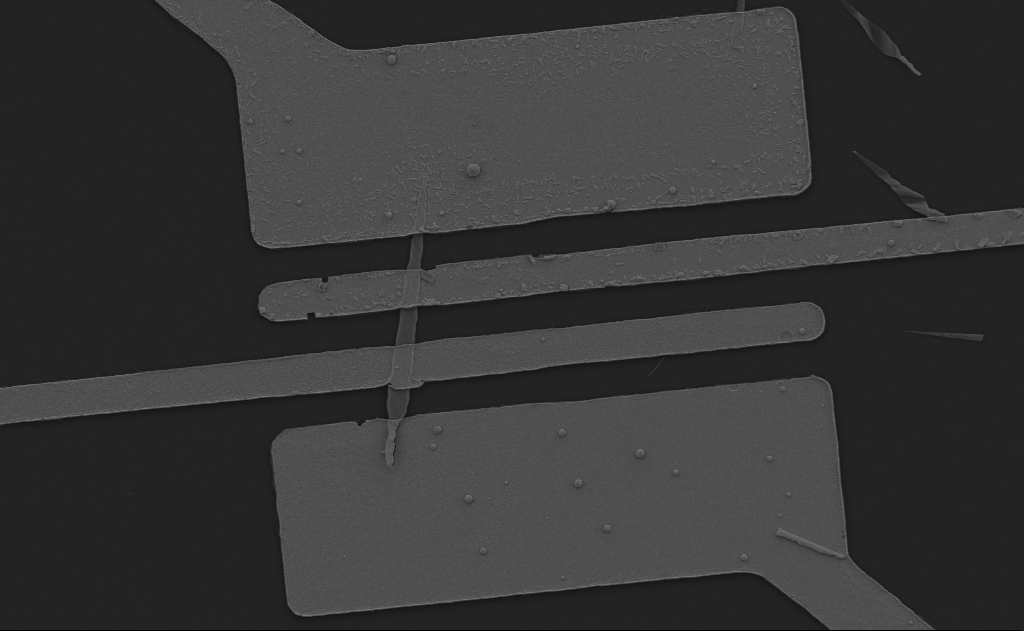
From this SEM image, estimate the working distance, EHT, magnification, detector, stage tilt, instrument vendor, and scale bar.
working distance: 12 mm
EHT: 5 kV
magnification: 6.86 K X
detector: SE2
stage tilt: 0°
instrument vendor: Zeiss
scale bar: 10000 nm